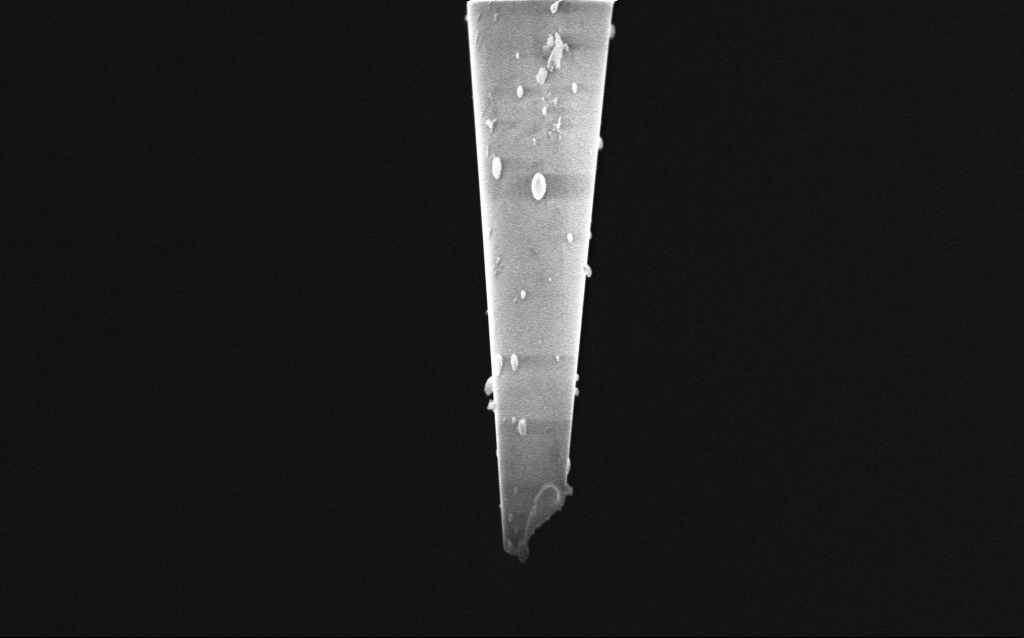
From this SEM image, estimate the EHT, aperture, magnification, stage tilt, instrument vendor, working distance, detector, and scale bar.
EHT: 5 kV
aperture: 30 µm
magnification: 10.4 K X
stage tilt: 45°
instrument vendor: Zeiss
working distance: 4 mm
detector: InLens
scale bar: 2000 nm